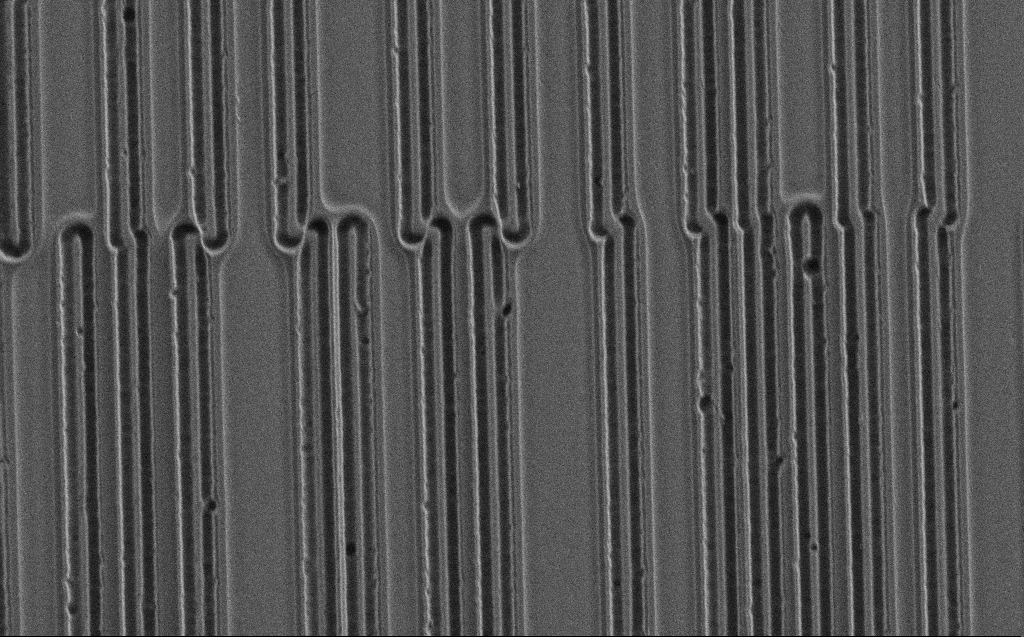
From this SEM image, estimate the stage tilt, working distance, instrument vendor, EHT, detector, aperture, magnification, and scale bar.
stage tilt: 0°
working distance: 4 mm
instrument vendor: Zeiss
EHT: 3 kV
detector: SE2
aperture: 30 µm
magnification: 3.16 K X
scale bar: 10000 nm